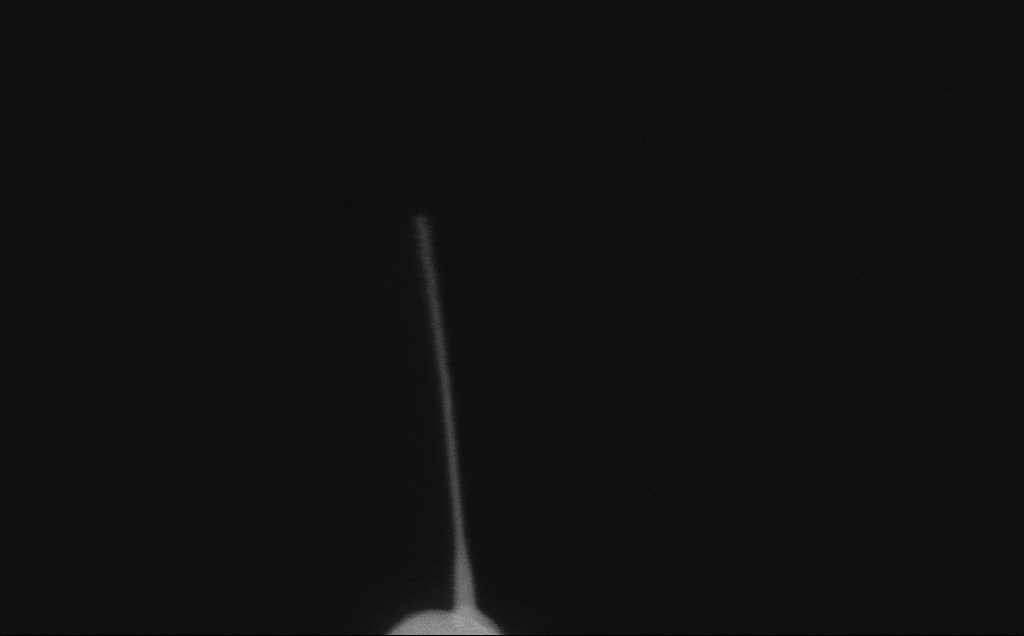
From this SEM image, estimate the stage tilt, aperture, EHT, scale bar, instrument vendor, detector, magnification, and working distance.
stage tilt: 0°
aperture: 30 µm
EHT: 10 kV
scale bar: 200 nm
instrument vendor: Zeiss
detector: InLens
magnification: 257.27 K X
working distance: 6 mm